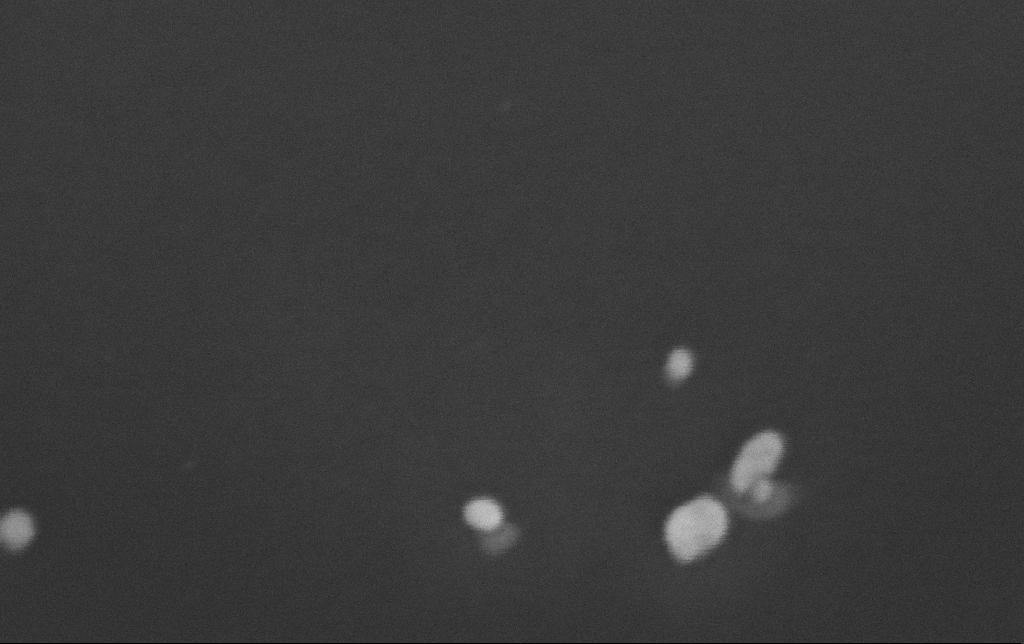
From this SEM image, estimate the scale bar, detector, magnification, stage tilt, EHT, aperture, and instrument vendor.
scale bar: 100 nm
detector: InLens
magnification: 500 K X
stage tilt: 45°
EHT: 10 kV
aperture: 30 µm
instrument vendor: Zeiss